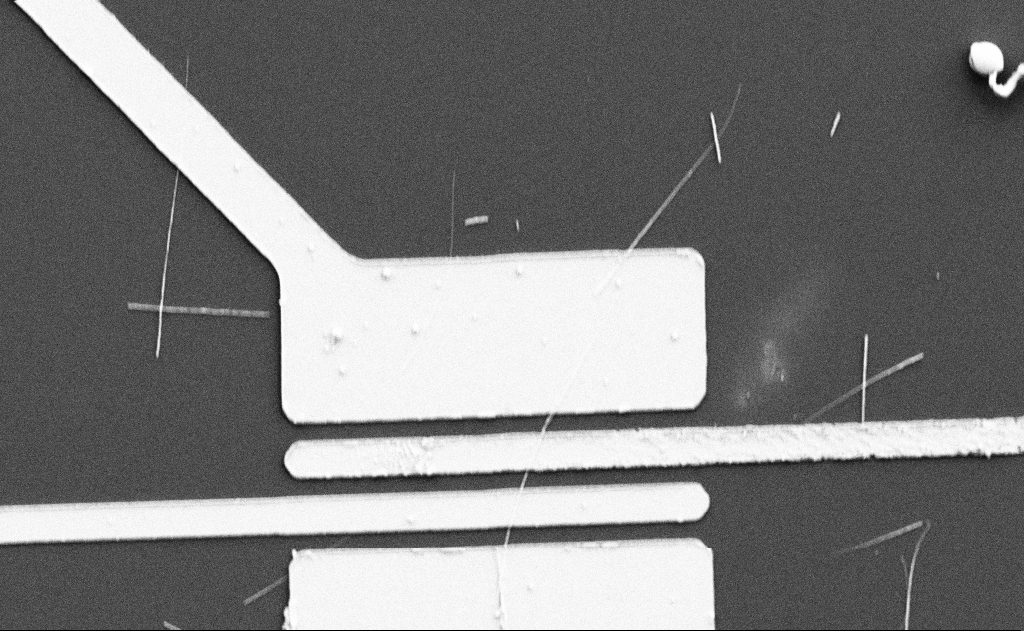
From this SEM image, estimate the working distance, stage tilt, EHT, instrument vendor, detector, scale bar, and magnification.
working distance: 16 mm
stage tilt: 0°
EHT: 5 kV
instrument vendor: Zeiss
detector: SE2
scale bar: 10000 nm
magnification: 5.19 K X